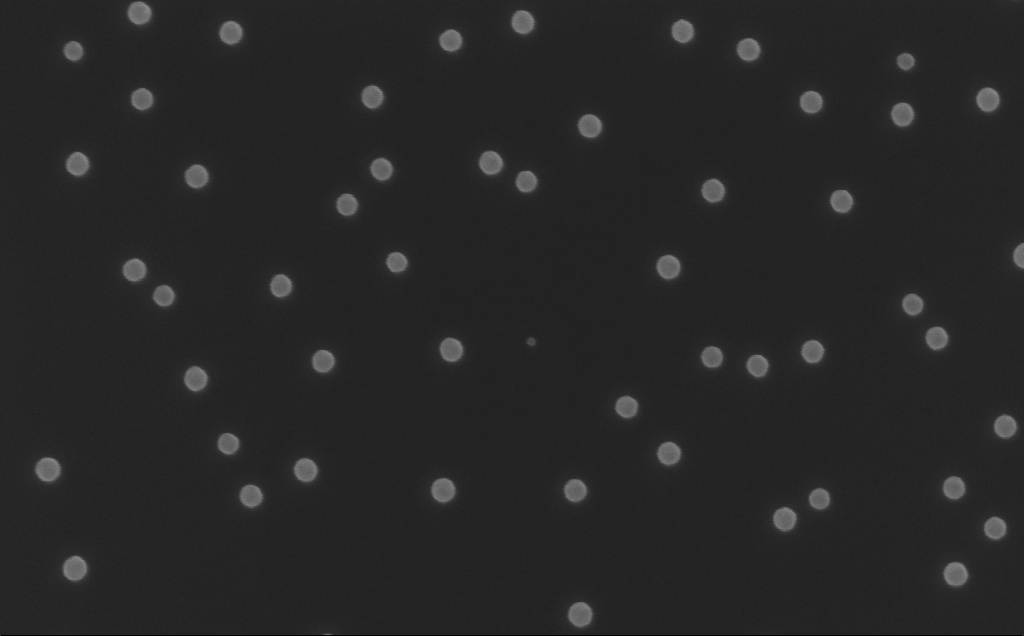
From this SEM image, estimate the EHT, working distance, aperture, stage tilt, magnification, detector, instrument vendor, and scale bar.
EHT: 10 kV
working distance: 8 mm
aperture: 30 µm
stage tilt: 0°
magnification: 150 K X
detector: InLens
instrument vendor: Zeiss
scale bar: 100 nm